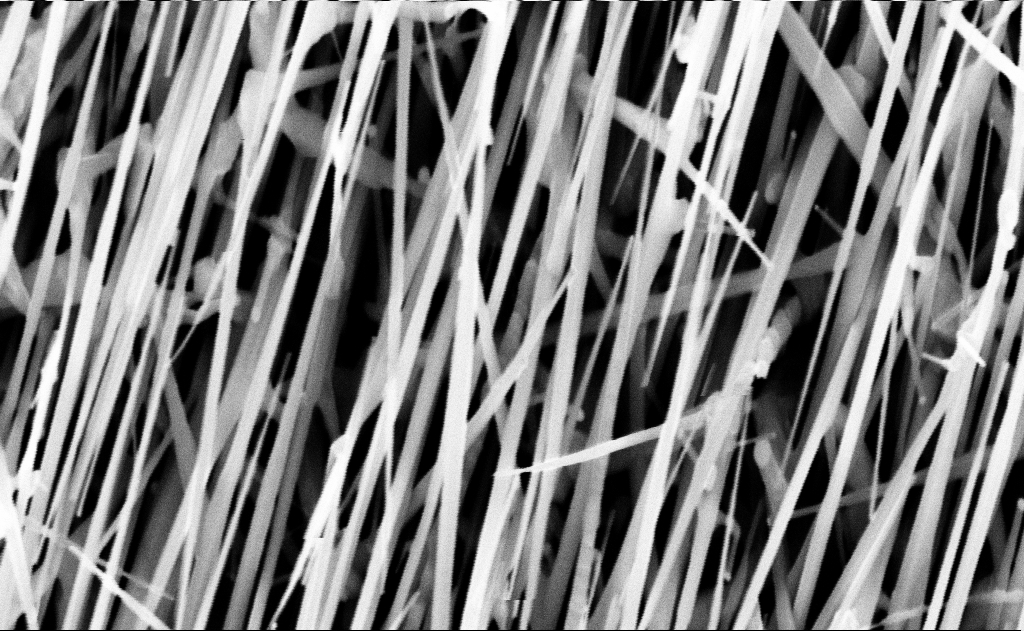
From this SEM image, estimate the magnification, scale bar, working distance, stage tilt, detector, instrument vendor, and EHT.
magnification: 60 K X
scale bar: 1000 nm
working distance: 16 mm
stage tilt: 0°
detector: InLens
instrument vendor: Zeiss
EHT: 10 kV